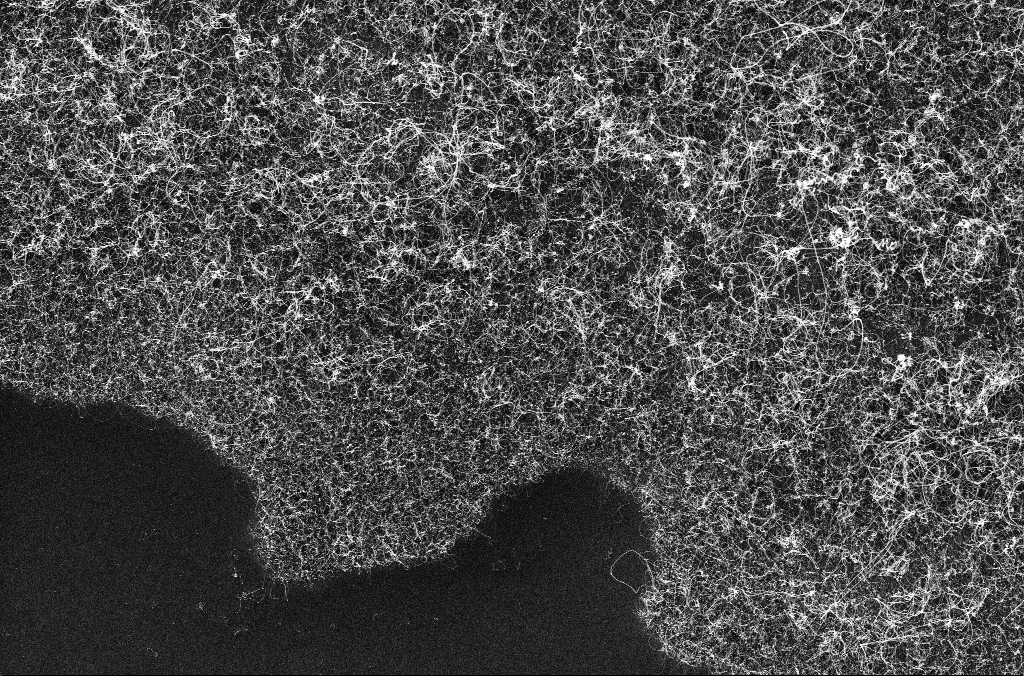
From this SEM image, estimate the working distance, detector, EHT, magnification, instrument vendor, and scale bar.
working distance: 3.3 mm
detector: InLens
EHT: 10 kV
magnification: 5 K X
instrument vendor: Zeiss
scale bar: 10000 nm